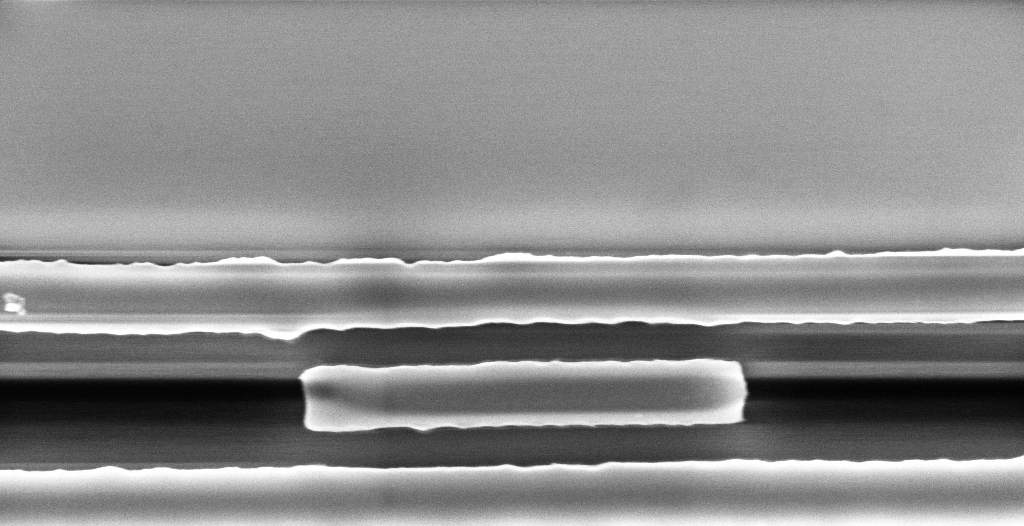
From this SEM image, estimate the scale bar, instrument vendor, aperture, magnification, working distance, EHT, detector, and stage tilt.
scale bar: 1000 nm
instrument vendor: Zeiss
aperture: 30 µm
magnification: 54.53 K X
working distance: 5.2 mm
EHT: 5 kV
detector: InLens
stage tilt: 0°